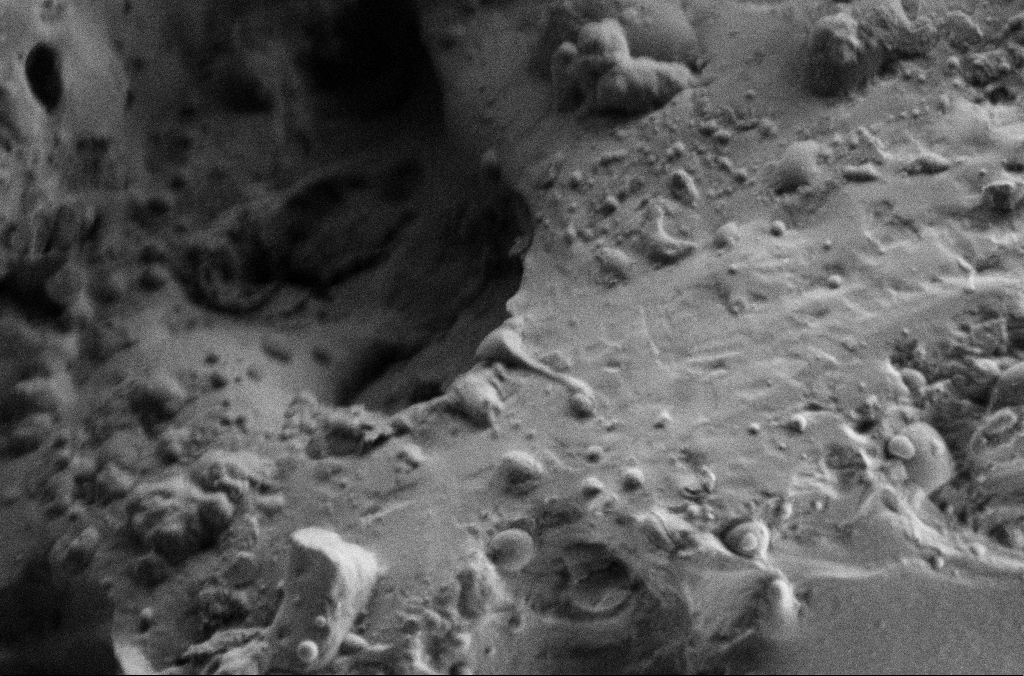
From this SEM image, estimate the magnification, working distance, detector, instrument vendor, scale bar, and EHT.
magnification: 10 K X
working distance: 3 mm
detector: SE2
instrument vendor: Zeiss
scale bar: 2000 nm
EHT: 2 kV